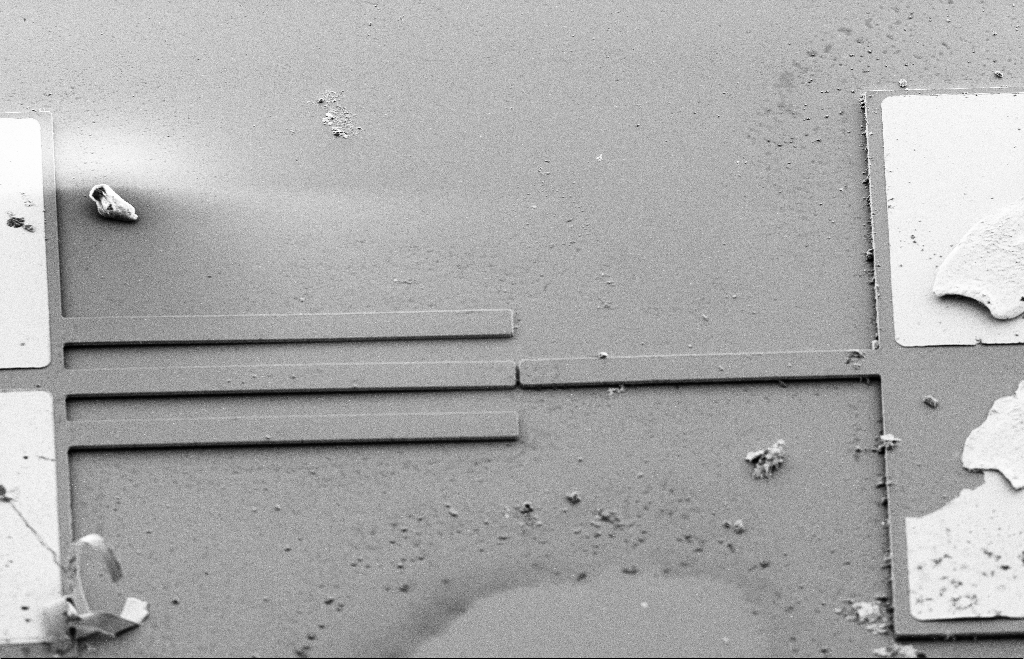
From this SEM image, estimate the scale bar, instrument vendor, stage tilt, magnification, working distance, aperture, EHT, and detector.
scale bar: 20000 nm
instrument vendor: Zeiss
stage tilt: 40.8°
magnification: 0.66 K X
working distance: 10 mm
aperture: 20 µm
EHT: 5 kV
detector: SE2